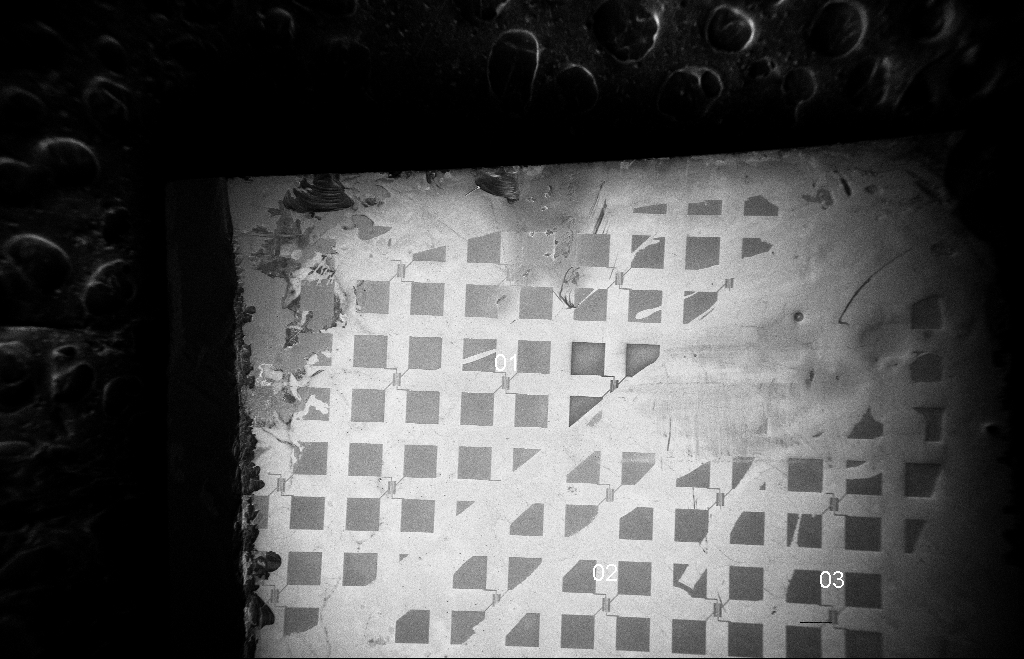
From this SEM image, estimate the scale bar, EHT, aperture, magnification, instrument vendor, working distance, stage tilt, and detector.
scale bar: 200000 nm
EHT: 5 kV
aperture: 20 µm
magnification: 0.083 K X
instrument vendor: Zeiss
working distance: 8 mm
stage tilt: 0°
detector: InLens